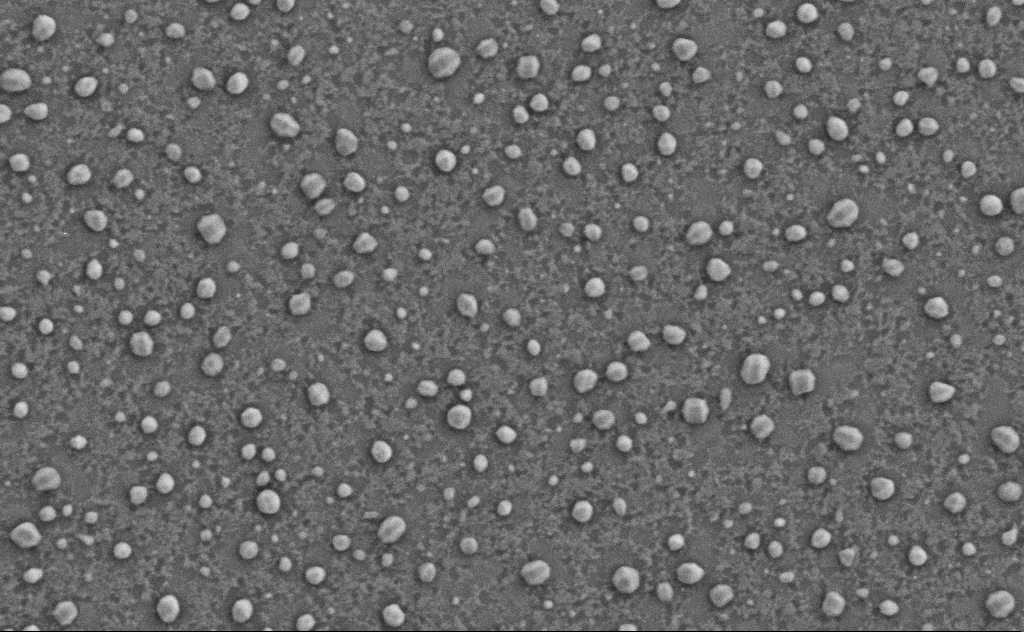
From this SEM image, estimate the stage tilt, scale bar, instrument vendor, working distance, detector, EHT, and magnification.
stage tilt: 0°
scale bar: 200 nm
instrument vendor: Zeiss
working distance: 4 mm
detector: SE2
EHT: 3 kV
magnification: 80 K X